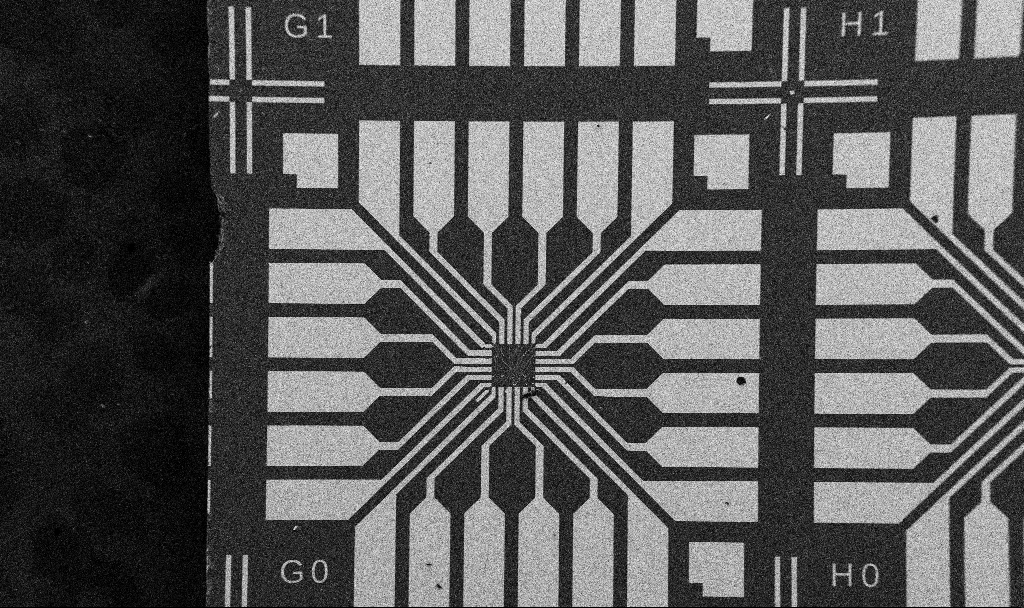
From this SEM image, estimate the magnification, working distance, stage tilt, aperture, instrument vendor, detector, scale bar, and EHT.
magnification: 0.1 K X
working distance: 10.7 mm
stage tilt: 0°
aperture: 30 µm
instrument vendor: Zeiss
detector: SE2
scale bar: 200000 nm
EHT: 5 kV